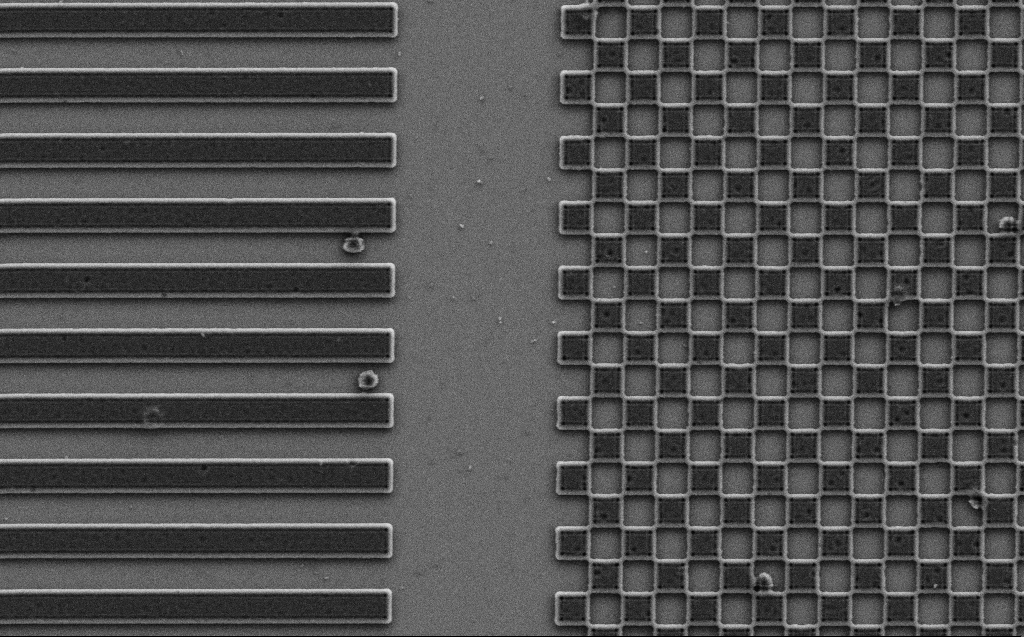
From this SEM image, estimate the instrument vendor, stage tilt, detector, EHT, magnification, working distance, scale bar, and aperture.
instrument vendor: Zeiss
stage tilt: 0°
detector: SE2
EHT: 3 kV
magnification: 12.13 K X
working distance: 6 mm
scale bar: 1000 nm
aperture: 30 µm